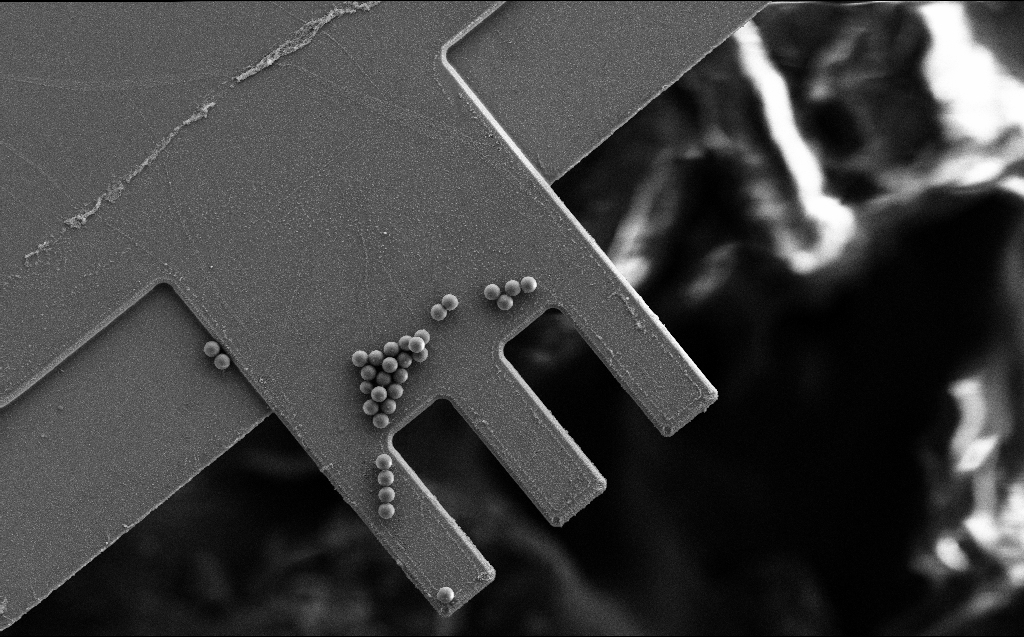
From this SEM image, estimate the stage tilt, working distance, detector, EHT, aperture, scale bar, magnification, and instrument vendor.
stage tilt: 0°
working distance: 5 mm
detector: SE2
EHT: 10 kV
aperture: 30 µm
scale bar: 10000 nm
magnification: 1.32 K X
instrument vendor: Zeiss